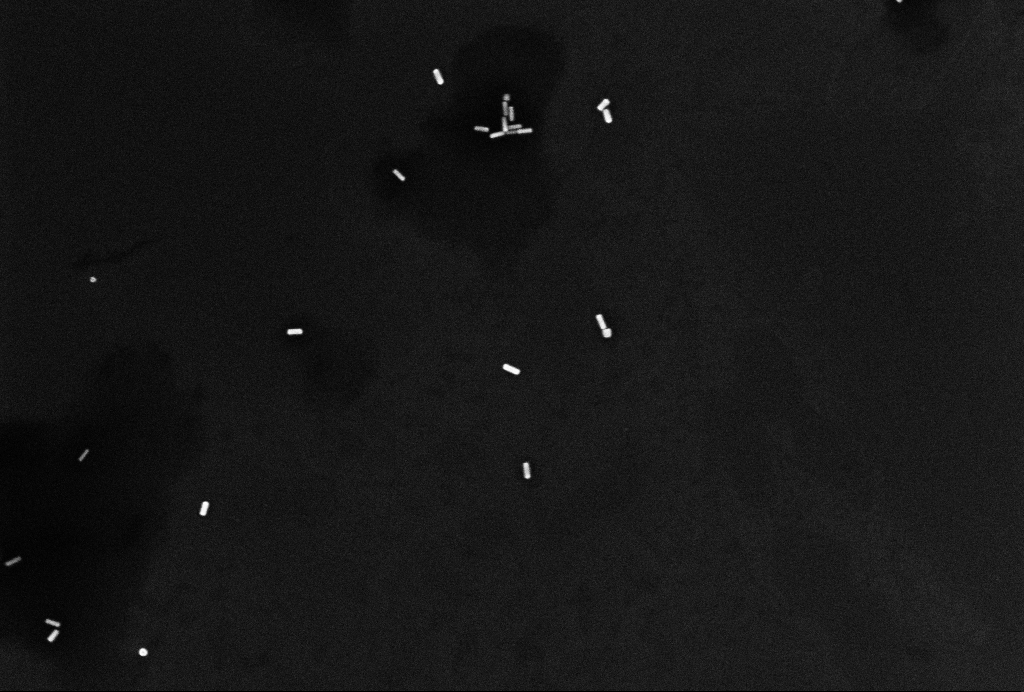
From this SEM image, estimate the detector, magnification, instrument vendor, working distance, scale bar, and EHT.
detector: InLens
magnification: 80 K X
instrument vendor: Zeiss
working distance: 3.2 mm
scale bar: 200 nm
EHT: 10 kV